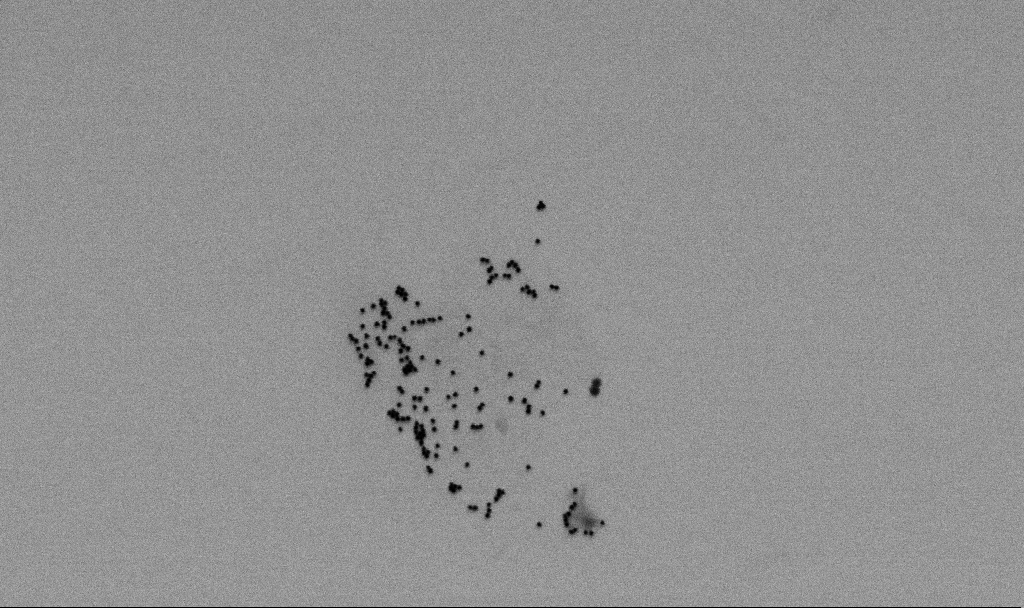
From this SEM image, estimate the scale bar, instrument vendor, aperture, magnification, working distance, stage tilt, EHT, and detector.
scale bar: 200 nm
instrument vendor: Zeiss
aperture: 30 µm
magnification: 75.26 K X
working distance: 6.5 mm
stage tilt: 0°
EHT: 2 kV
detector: SE2